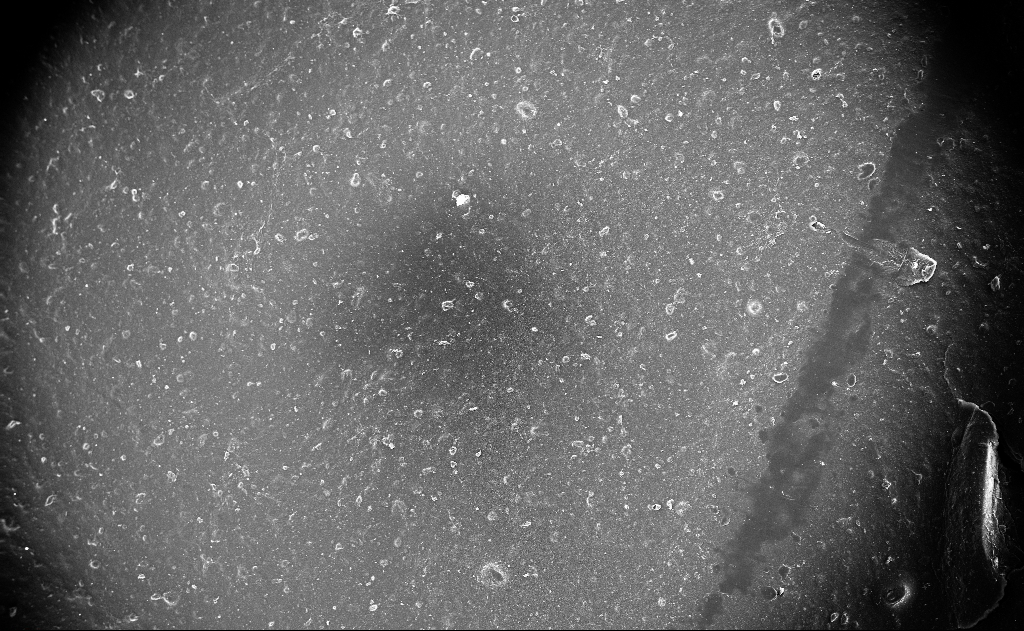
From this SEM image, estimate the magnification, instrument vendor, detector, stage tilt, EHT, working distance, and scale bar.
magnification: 0.129 K X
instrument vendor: Zeiss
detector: InLens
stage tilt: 0°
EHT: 10 kV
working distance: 5 mm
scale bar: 100000 nm